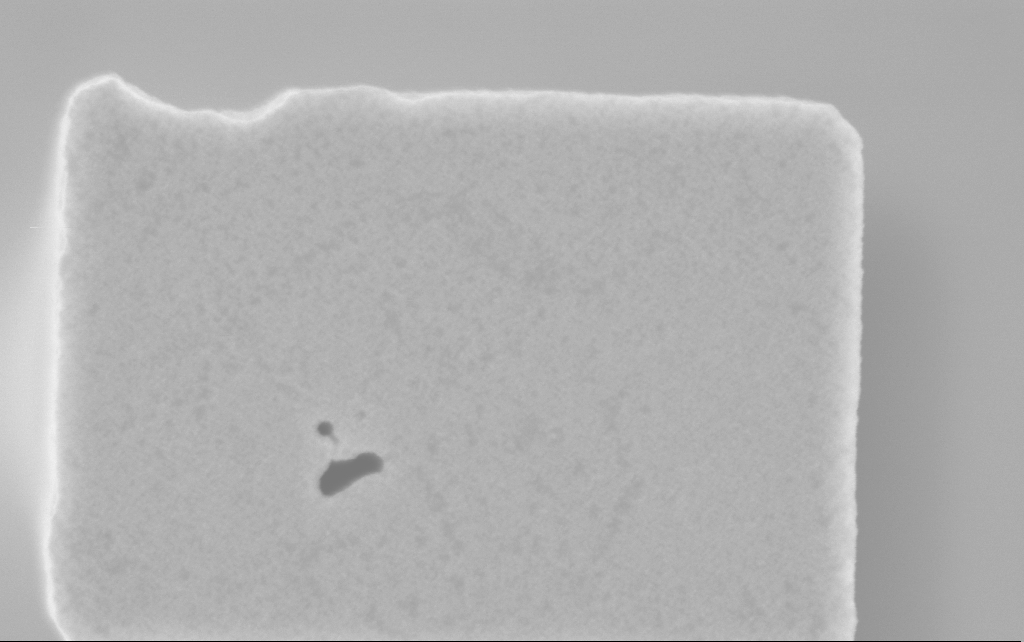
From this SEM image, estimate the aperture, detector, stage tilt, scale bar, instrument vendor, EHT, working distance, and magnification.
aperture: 30 µm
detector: InLens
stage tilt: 0°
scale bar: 200 nm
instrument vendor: Zeiss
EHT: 3 kV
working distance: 2.5 mm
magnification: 97.96 K X